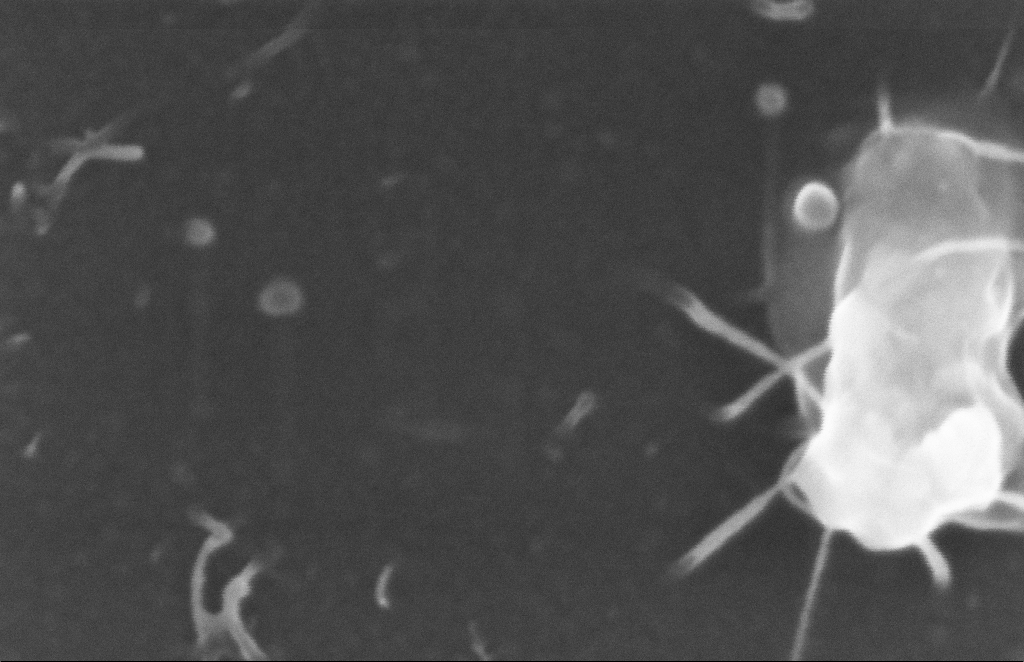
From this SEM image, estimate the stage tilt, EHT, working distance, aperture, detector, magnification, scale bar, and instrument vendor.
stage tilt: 0°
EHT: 5 kV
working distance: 8 mm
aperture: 20 µm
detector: InLens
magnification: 399.53 K X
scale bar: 100 nm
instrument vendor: Zeiss